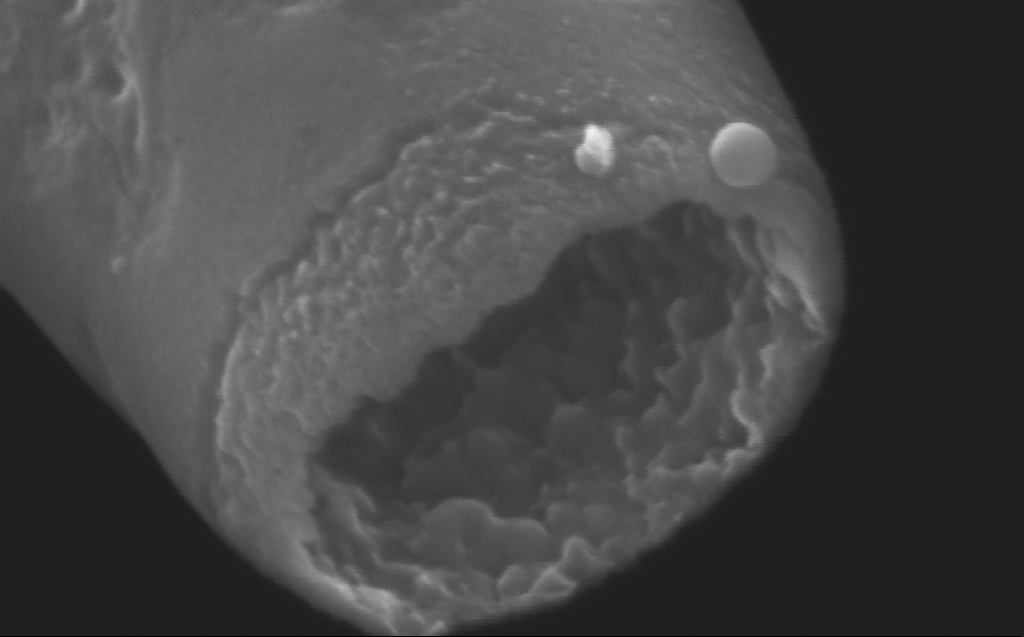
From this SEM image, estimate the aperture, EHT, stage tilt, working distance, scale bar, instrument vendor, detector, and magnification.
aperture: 30 µm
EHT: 5 kV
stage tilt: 45°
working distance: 4 mm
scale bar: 200 nm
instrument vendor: Zeiss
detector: InLens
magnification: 339.22 K X